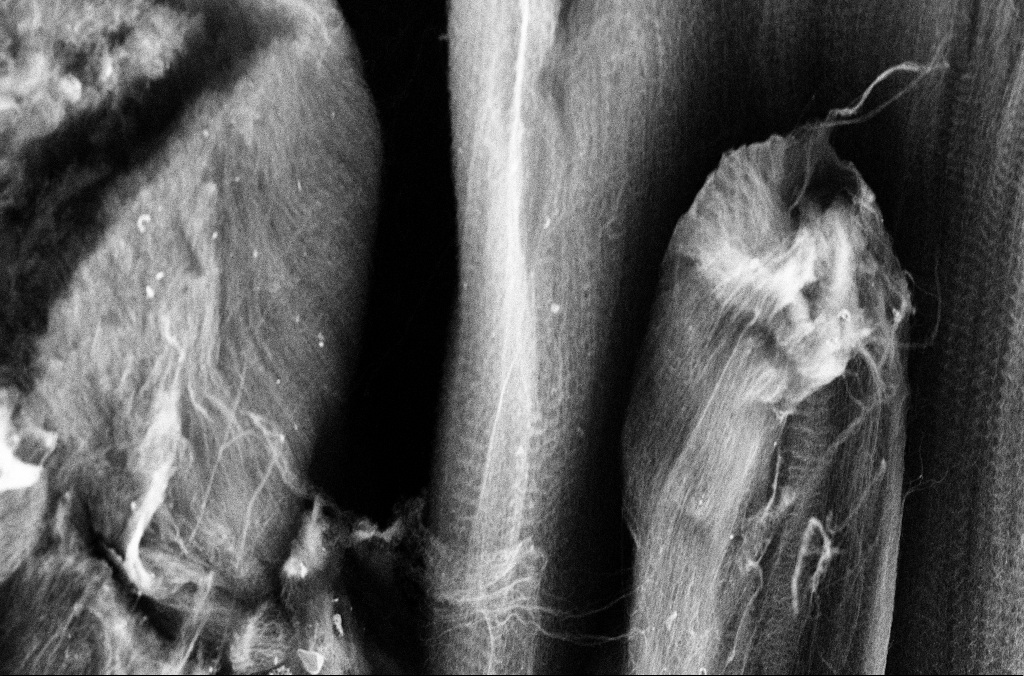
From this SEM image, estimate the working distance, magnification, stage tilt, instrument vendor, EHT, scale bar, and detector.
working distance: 3.4 mm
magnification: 5 K X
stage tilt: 45°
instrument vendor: Zeiss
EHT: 3 kV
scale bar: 10000 nm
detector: SE2